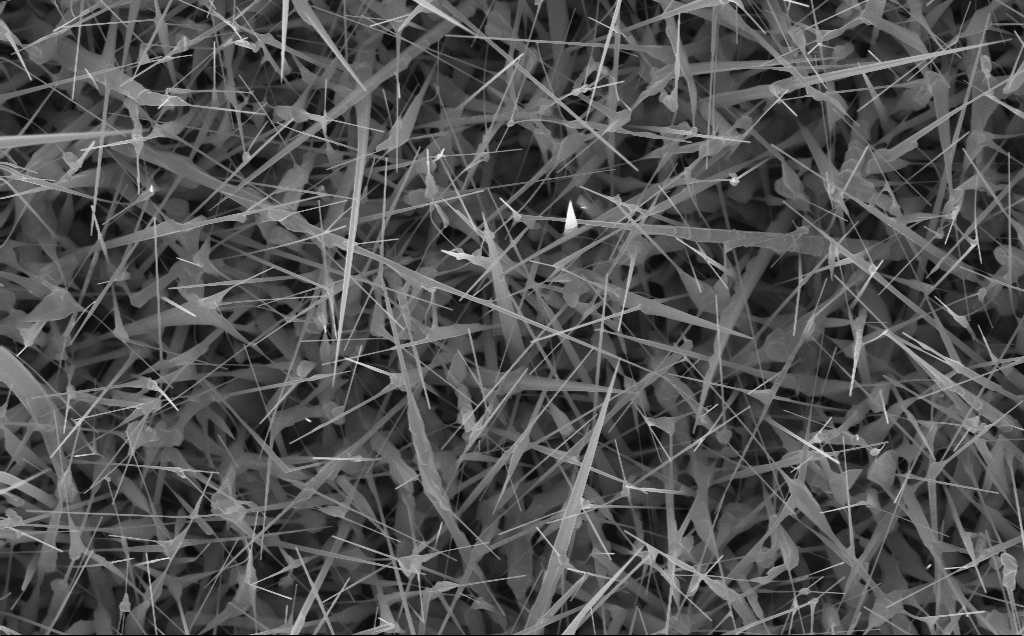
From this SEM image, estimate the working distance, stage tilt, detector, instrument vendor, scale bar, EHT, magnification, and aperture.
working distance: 4 mm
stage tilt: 0°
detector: InLens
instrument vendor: Zeiss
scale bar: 2000 nm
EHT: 10 kV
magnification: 20 K X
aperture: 30 µm